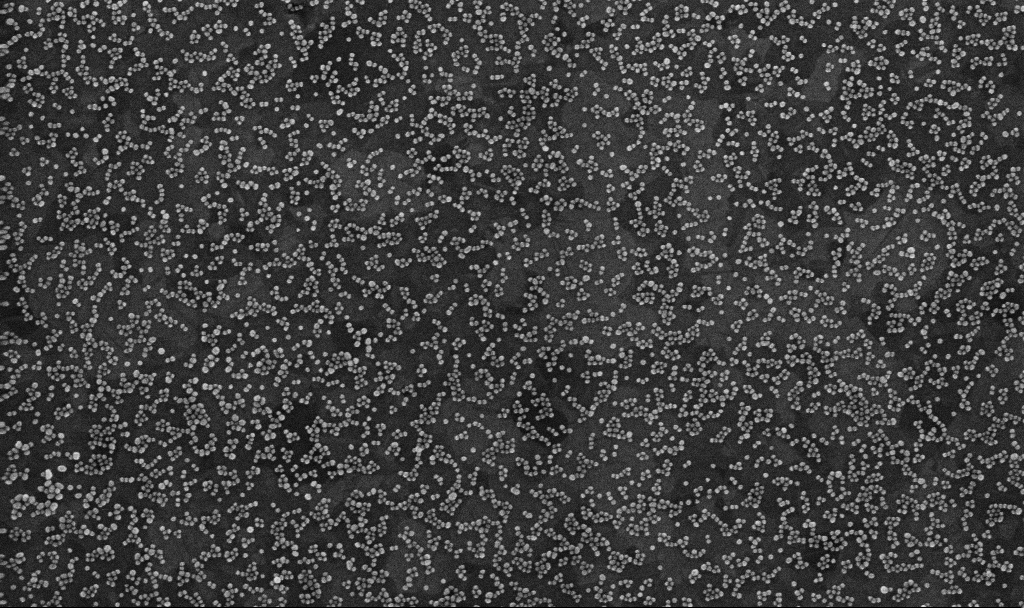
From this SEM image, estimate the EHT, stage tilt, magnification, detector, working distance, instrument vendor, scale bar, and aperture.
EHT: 10 kV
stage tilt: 0°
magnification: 100 K X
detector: InLens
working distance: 3.3 mm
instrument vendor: Zeiss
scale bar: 200 nm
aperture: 30 µm